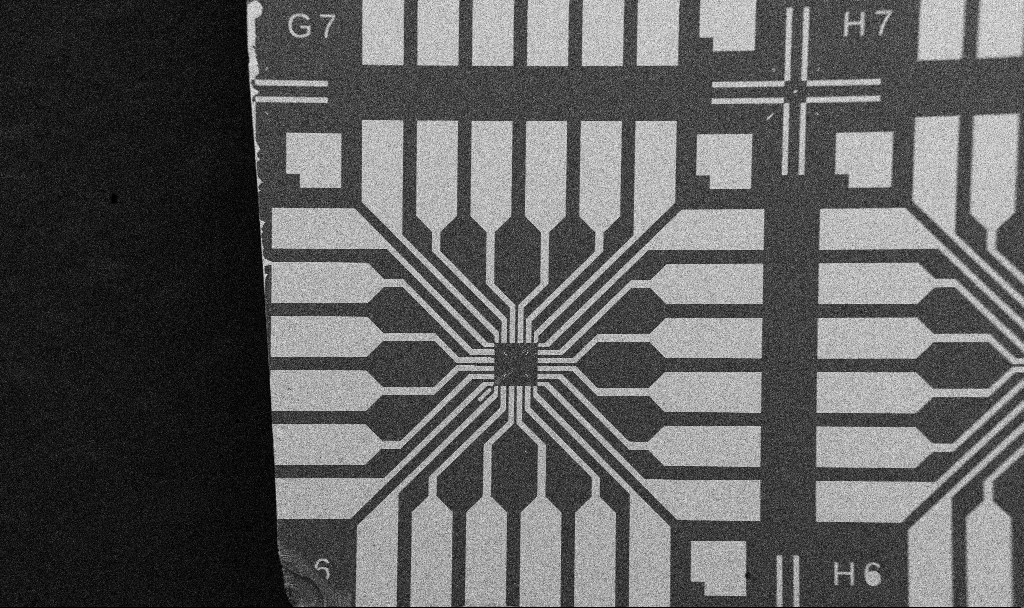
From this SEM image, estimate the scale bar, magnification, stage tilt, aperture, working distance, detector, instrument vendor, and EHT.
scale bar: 200000 nm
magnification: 0.1 K X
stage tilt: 0°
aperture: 30 µm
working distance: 10.7 mm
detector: SE2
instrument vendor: Zeiss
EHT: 5 kV